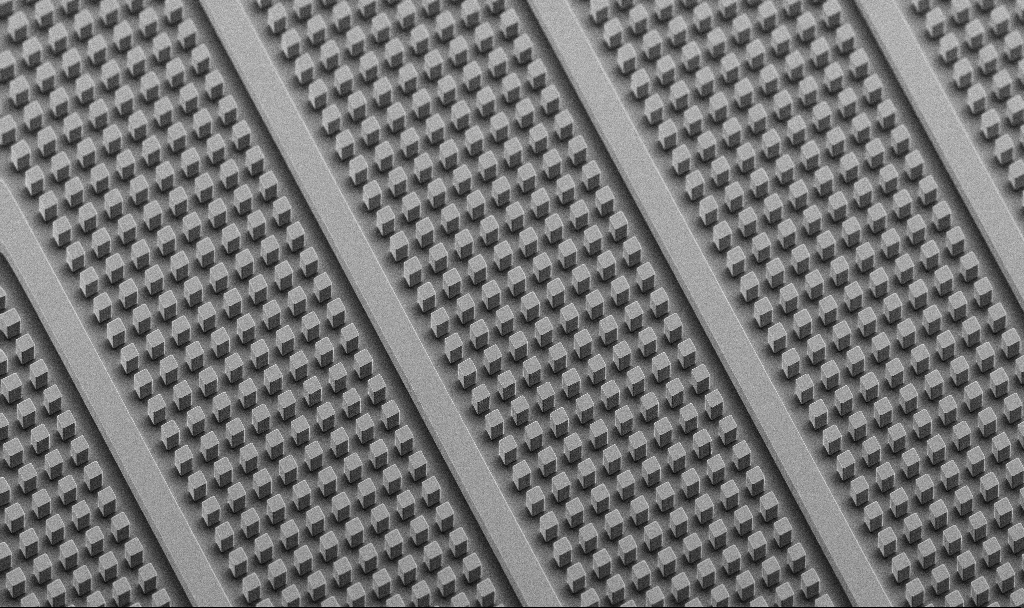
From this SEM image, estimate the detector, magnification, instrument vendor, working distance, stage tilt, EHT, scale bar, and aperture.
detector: SE2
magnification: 0.534 K X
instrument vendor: Zeiss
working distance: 8.2 mm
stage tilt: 45°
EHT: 5 kV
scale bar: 100000 nm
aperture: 30 µm